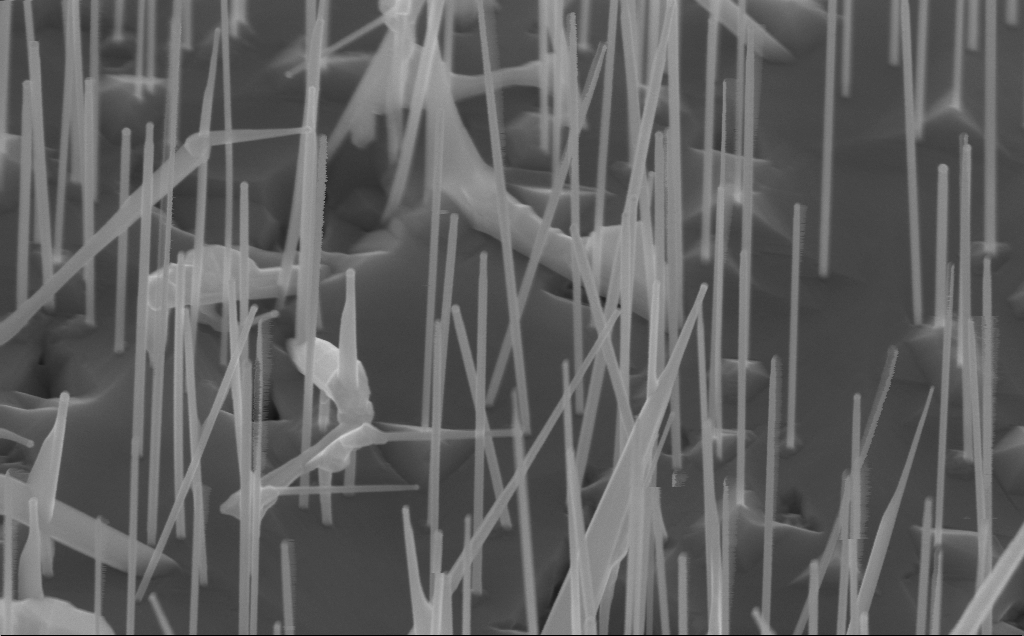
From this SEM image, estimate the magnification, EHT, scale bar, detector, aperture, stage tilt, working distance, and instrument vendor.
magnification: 80 K X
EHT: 10 kV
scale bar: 200 nm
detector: InLens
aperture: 30 µm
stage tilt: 45°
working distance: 5 mm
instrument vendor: Zeiss